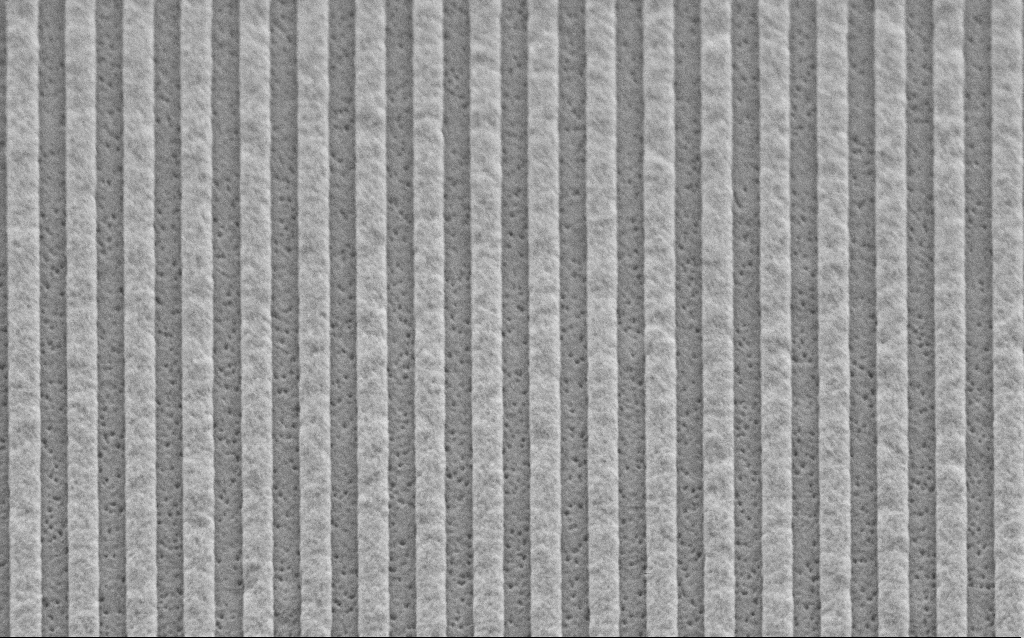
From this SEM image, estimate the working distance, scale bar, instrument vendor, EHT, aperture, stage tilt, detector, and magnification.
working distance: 6.6 mm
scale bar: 1000 nm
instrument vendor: Zeiss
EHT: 3 kV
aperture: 30 µm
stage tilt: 45°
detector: SE2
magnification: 51.99 K X